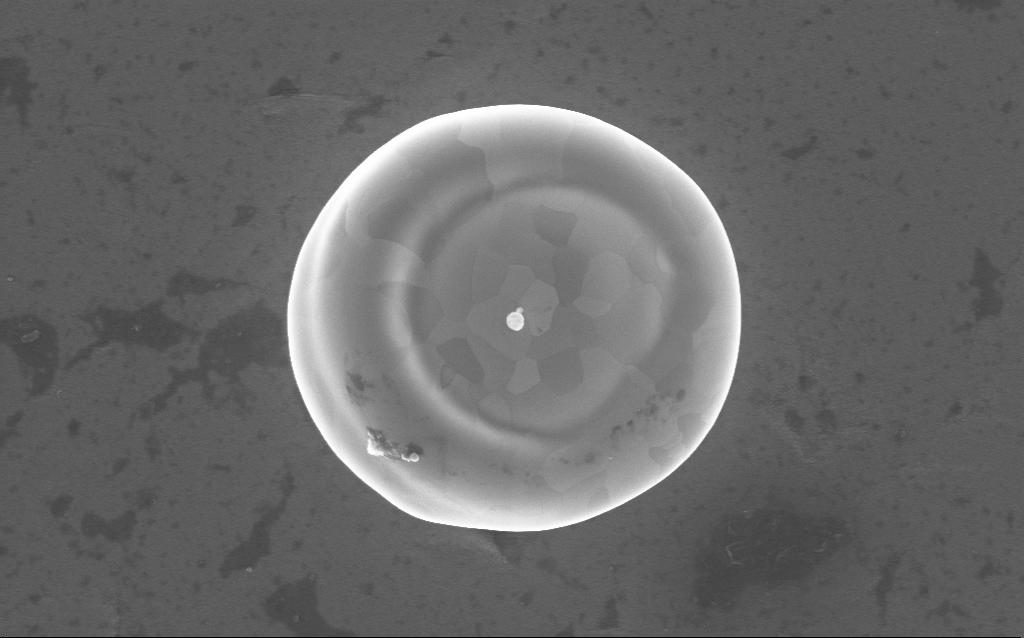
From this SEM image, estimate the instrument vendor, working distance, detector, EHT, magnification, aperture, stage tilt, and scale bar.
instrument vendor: Zeiss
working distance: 4 mm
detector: InLens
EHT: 5 kV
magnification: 36.96 K X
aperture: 30 µm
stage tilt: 0°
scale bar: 1000 nm